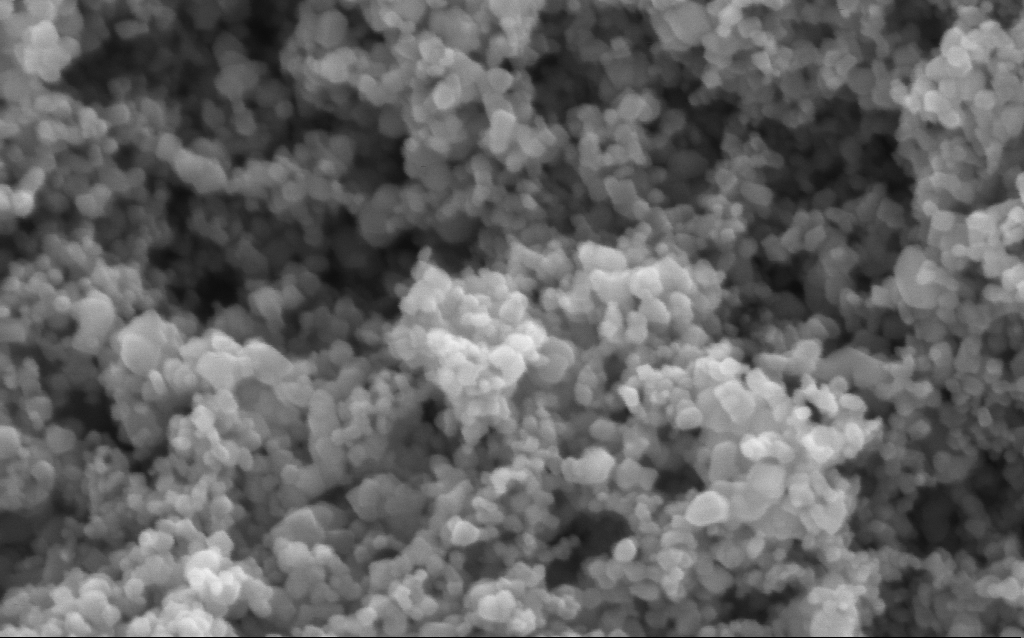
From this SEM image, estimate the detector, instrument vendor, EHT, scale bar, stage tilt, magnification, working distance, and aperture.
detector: InLens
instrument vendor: Zeiss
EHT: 5 kV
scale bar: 200 nm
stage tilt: -0°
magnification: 294.04 K X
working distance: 4.3 mm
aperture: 30 µm